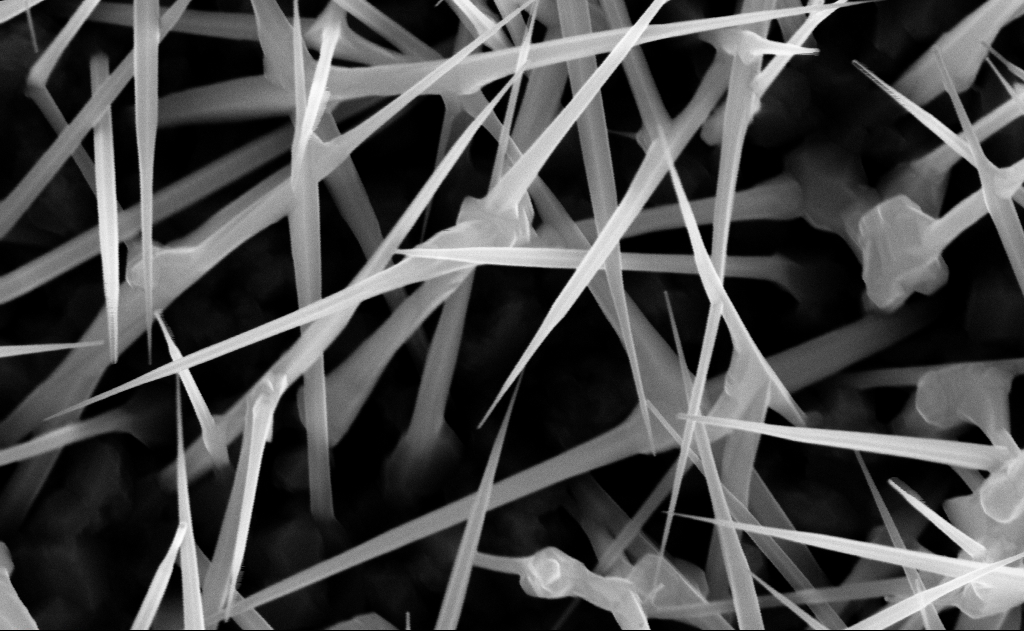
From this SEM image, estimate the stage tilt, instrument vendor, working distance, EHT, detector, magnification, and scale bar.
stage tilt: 0°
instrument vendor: Zeiss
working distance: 9 mm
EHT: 10 kV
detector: InLens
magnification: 100 K X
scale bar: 200 nm